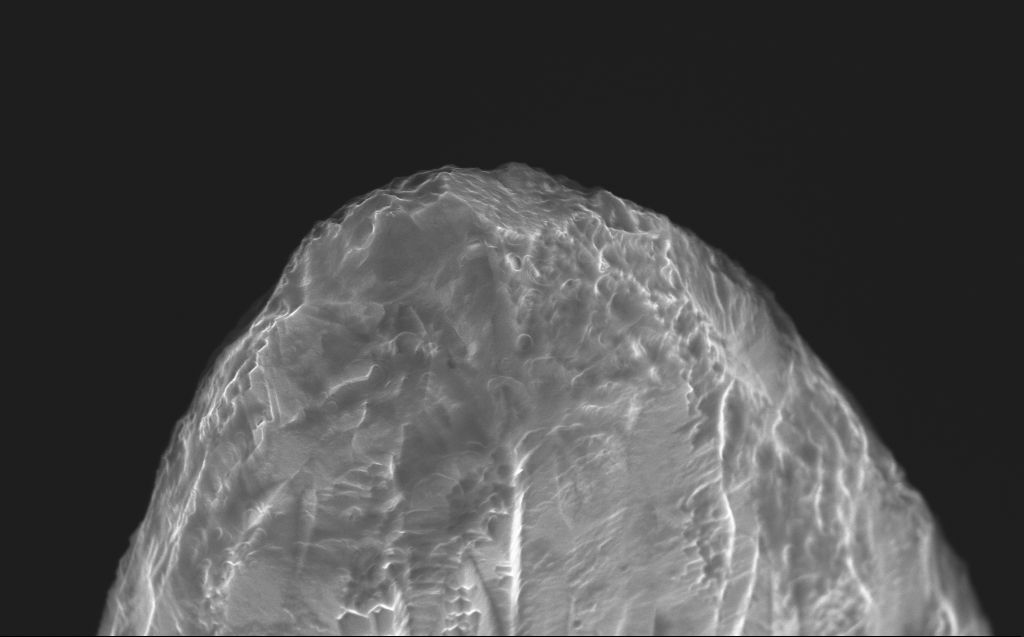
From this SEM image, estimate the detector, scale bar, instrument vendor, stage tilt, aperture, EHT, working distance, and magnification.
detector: InLens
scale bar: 1000 nm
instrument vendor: Zeiss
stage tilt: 40°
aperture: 30 µm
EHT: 10 kV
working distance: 4 mm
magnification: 53.53 K X